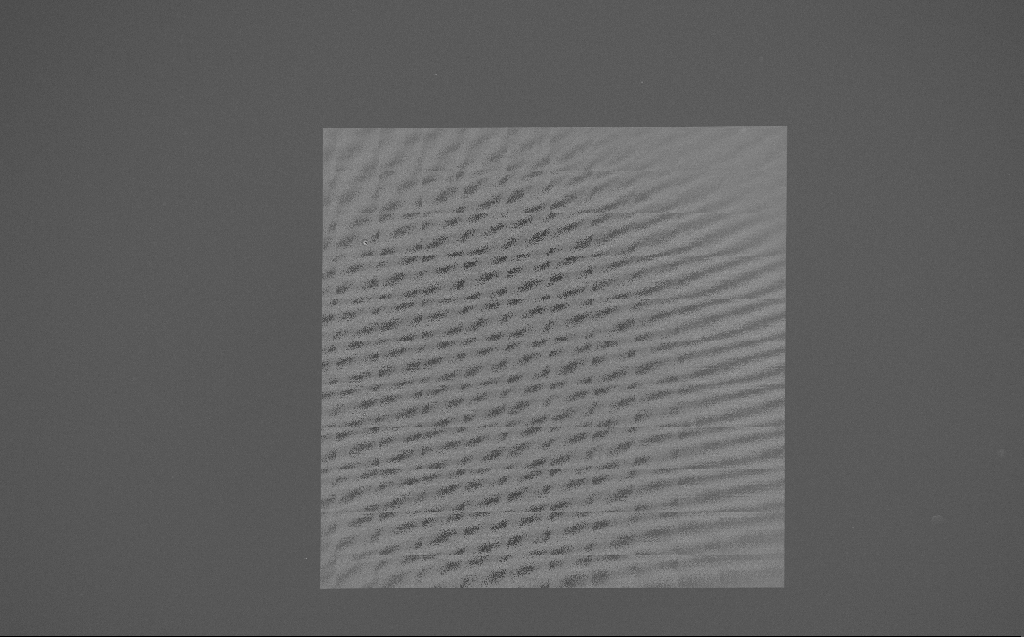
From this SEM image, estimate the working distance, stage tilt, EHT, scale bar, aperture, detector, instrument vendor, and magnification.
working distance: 7 mm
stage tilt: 0°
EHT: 5 kV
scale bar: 100000 nm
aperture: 30 µm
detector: InLens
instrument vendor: Zeiss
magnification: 0.231 K X